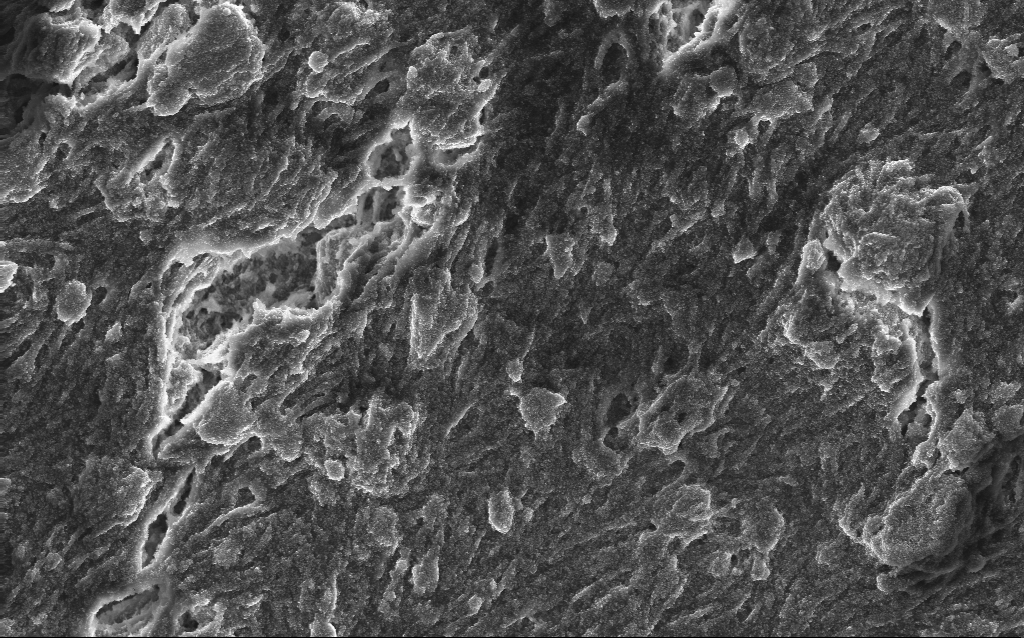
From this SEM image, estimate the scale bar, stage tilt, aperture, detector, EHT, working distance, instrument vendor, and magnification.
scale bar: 10000 nm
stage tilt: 0°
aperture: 30 µm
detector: InLens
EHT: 10 kV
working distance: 2.6 mm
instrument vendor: Zeiss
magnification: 5.83 K X